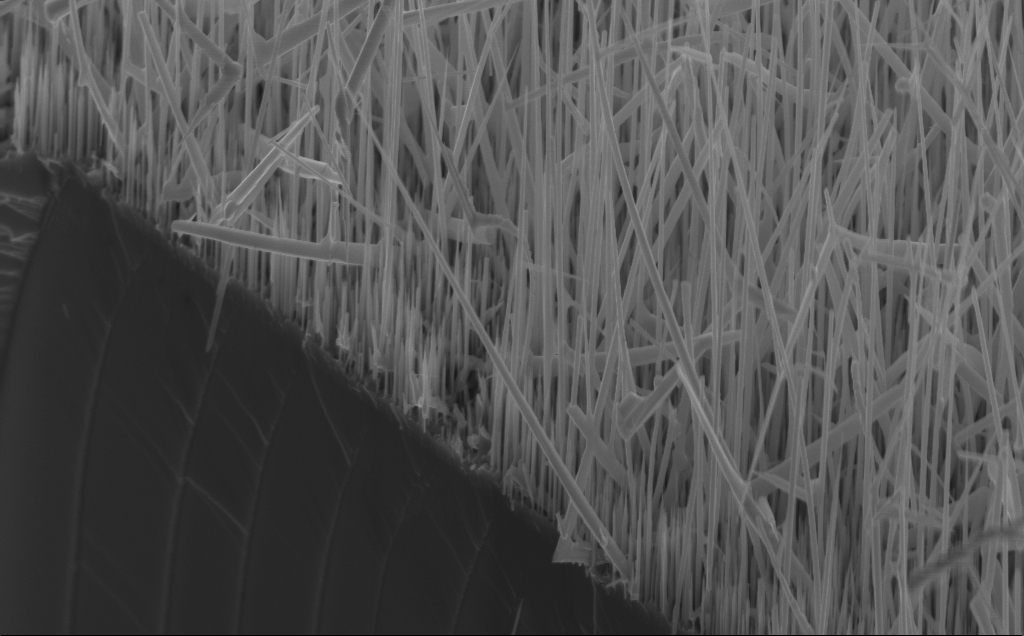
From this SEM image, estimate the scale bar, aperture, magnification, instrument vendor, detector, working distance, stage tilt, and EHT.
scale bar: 1000 nm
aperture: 30 µm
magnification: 17.4 K X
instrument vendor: Zeiss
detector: InLens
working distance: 5 mm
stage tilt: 45°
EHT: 10 kV